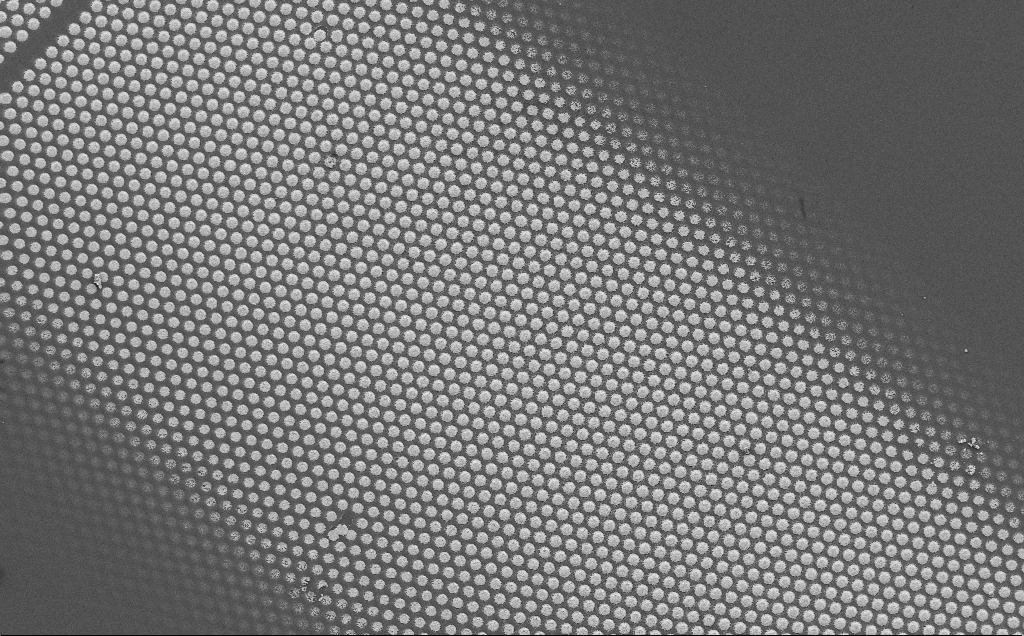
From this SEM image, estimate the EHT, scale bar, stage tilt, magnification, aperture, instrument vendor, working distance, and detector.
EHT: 5 kV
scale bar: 10000 nm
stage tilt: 0°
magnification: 1.95 K X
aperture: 30 µm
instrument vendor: Zeiss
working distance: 15 mm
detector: SE2